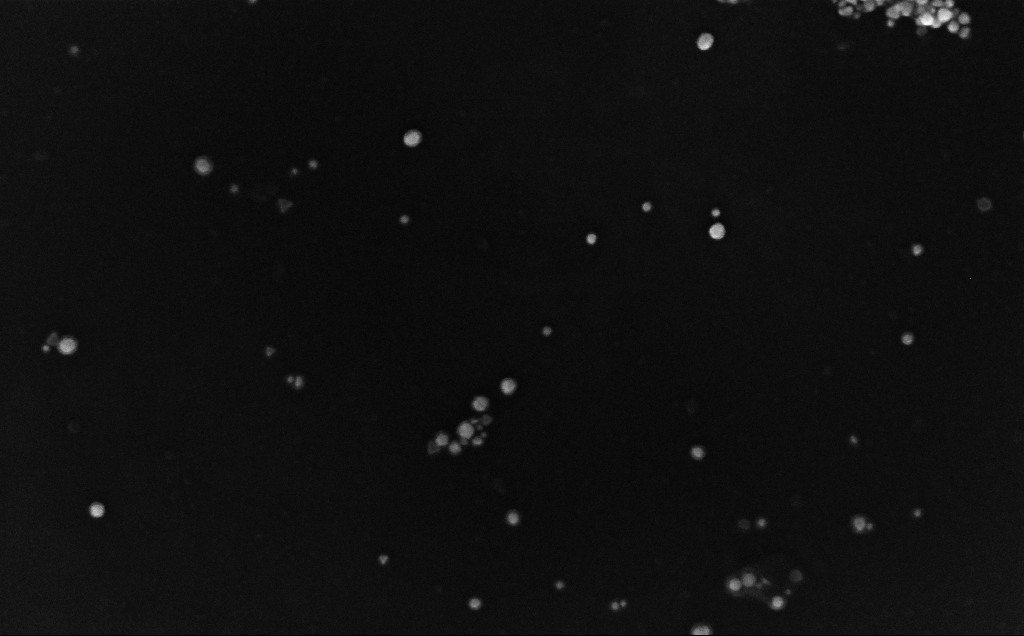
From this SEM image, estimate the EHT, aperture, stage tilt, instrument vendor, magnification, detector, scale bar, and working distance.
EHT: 10 kV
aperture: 30 µm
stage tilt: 0°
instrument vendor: Zeiss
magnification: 212.25 K X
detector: InLens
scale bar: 200 nm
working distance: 4 mm